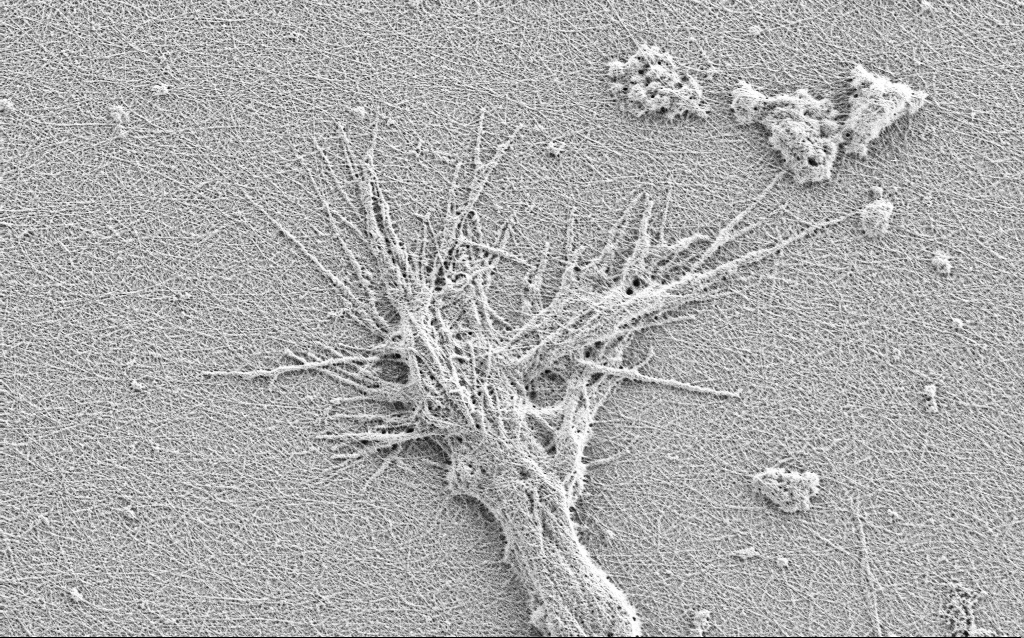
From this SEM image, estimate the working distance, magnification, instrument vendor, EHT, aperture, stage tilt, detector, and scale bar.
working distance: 3 mm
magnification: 10 K X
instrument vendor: Zeiss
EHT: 0.9 kV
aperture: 30 µm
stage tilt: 0°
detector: SE2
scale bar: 2000 nm